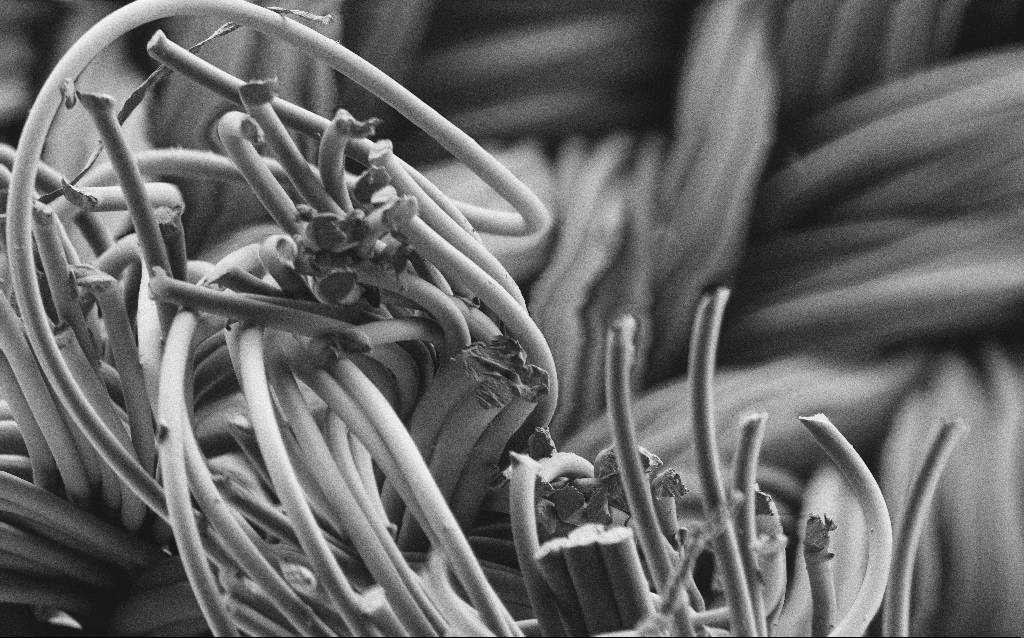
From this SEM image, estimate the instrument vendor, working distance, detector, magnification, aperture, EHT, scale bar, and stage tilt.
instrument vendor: Zeiss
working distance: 3 mm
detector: SE2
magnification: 0.379 K X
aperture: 30 µm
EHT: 1 kV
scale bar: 100000 nm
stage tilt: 0°